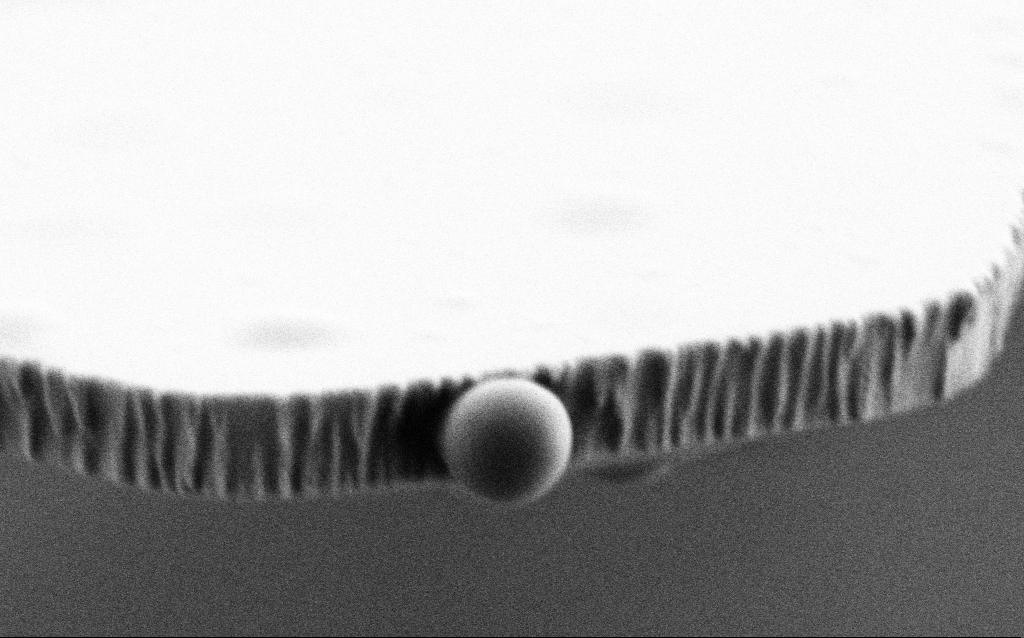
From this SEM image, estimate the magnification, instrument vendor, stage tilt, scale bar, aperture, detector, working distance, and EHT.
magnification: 50.6 K X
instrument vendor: Zeiss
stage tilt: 70°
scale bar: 1000 nm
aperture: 30 µm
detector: SE2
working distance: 6 mm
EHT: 3 kV